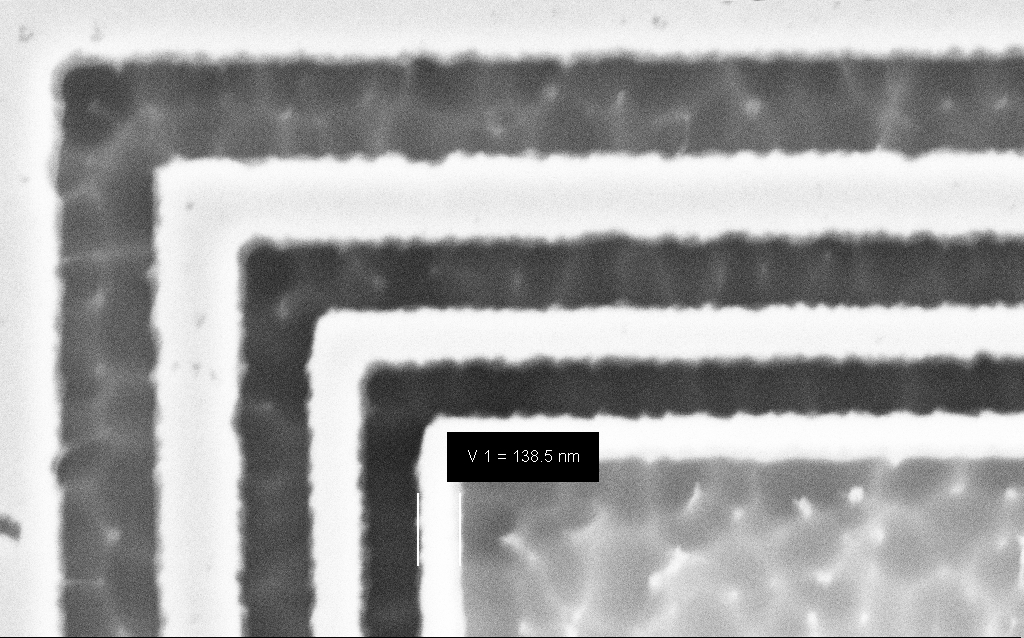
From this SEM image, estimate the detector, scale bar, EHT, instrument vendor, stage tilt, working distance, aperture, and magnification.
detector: SE2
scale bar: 200 nm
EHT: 3 kV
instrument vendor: Zeiss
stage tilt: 0°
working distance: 8 mm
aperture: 30 µm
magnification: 111.32 K X